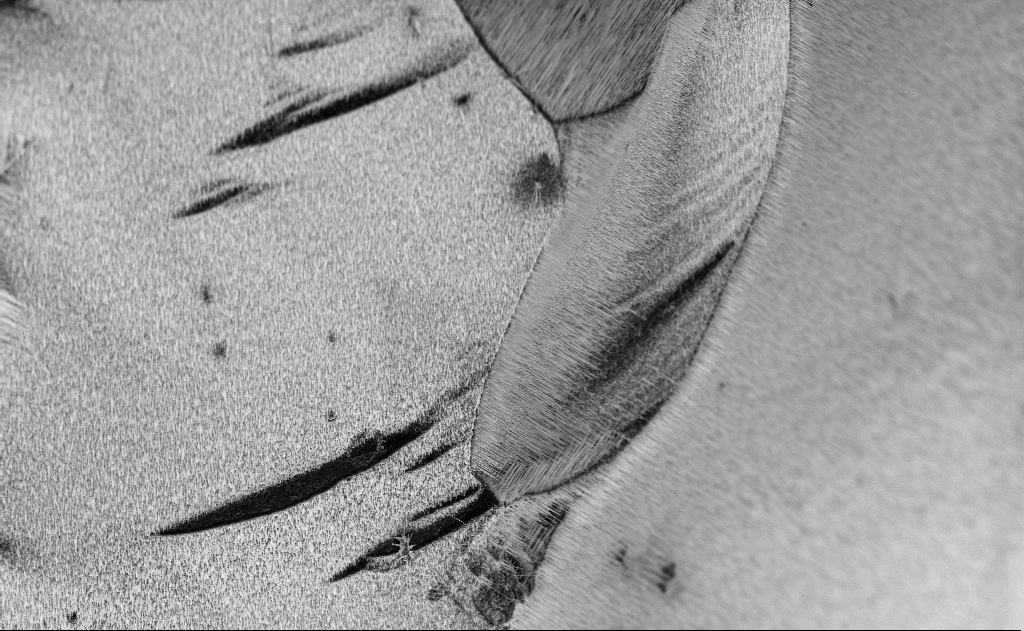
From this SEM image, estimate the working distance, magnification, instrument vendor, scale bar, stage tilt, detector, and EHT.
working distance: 6 mm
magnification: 1.58 K X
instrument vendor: Zeiss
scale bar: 10000 nm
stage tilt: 0°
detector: InLens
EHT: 10 kV